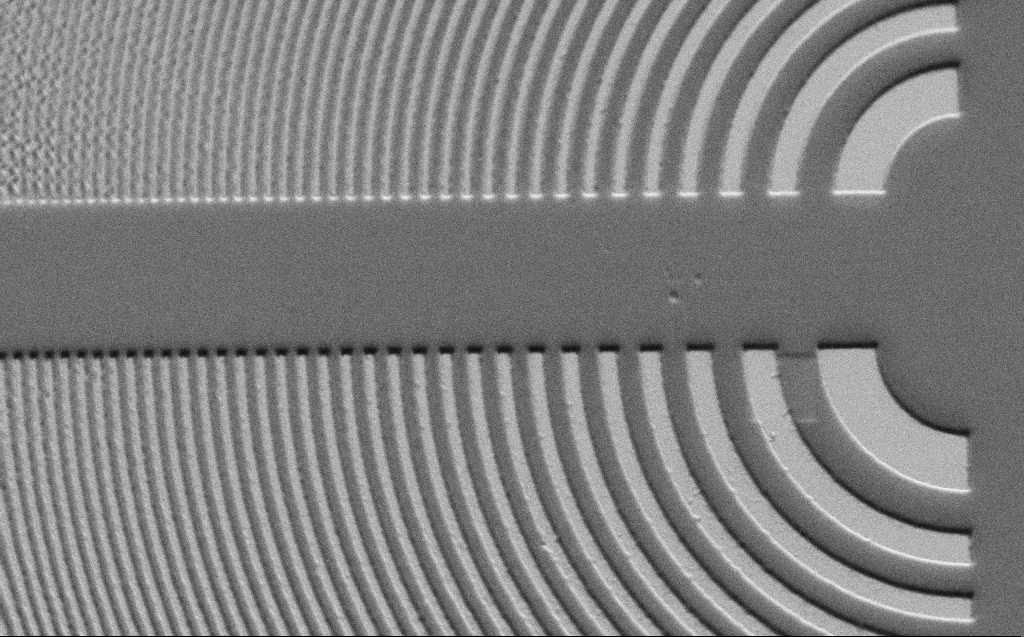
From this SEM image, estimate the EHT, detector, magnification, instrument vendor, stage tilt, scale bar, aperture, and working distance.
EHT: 3 kV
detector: SE2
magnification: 5.72 K X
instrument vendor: Zeiss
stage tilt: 45°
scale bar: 10000 nm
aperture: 30 µm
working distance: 6 mm